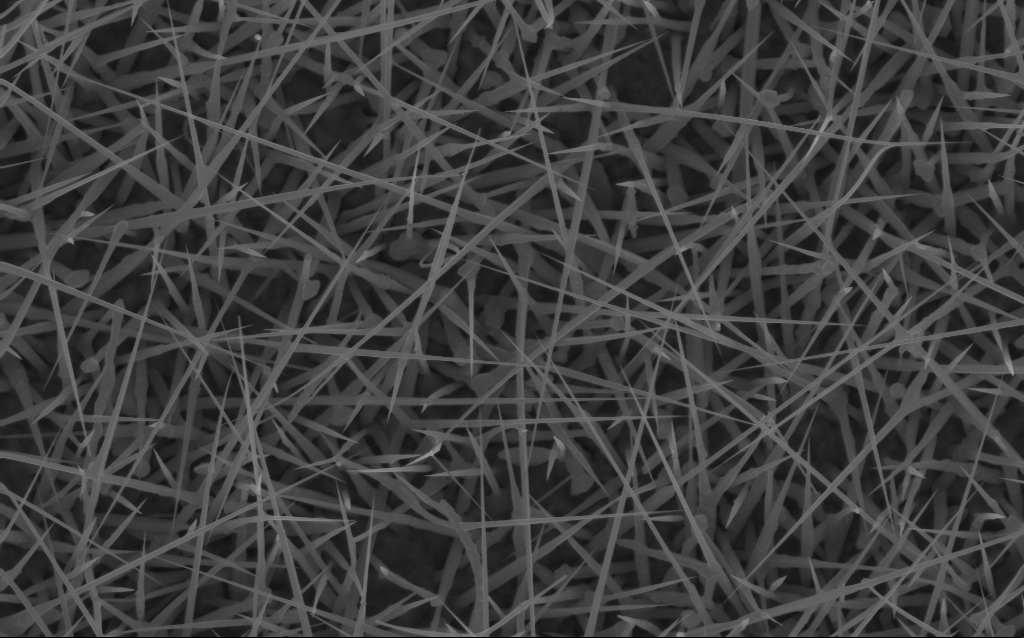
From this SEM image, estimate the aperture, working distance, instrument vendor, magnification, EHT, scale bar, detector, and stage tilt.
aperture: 30 µm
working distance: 7 mm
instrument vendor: Zeiss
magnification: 20 K X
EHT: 10 kV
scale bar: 2000 nm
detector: InLens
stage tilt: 0°